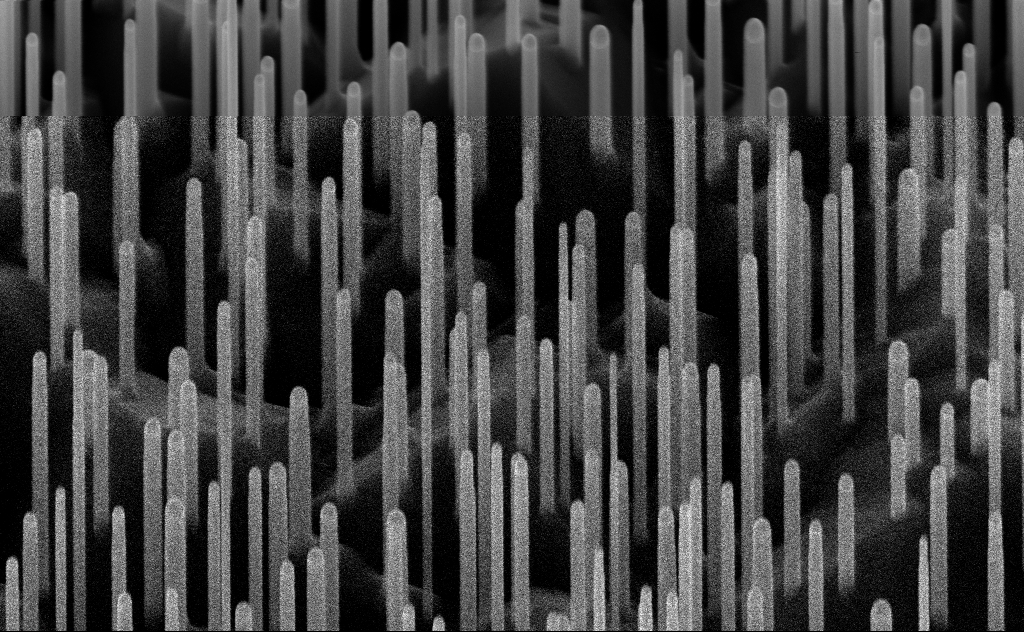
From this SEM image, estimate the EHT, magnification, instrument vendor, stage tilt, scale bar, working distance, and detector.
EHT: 10 kV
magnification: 80 K X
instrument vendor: Zeiss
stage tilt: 45°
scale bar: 200 nm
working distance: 7 mm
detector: InLens